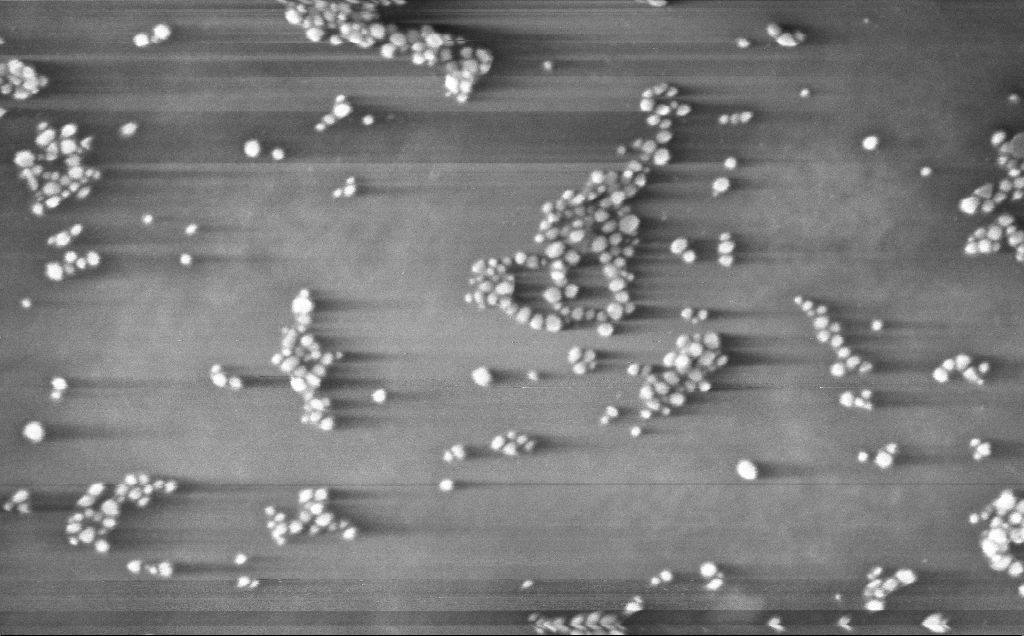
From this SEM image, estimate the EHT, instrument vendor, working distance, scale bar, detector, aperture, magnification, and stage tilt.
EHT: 10 kV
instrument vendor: Zeiss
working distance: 4 mm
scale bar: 200 nm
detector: InLens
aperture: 30 µm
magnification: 233.38 K X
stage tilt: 0°